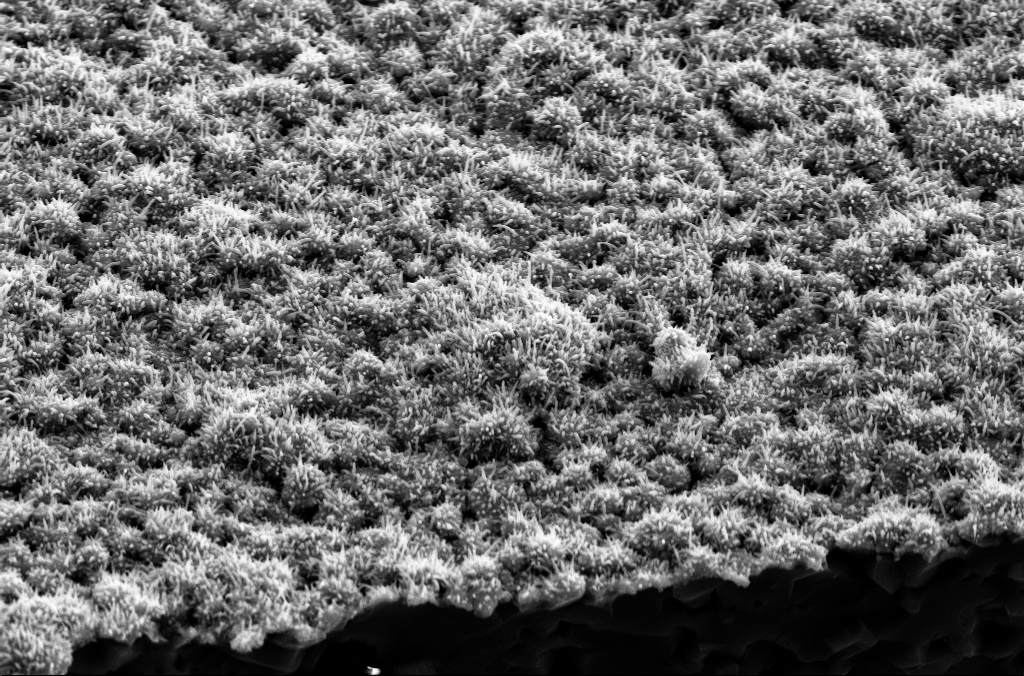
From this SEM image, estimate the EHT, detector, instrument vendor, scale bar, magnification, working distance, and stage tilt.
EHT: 10 kV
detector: SE2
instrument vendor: Zeiss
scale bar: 2000 nm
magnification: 17.52 K X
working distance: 8 mm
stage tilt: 45°